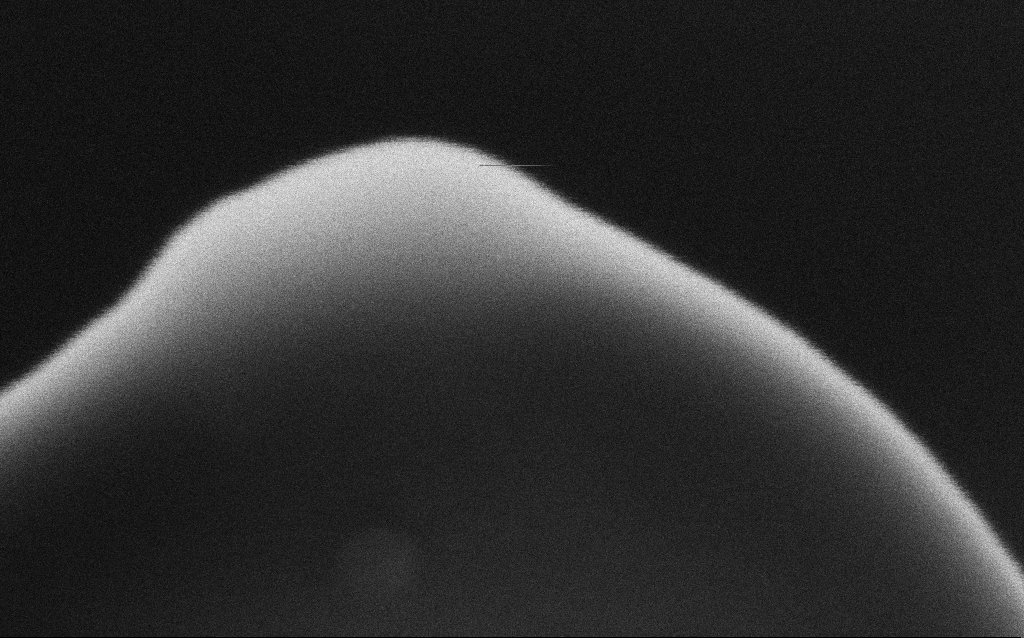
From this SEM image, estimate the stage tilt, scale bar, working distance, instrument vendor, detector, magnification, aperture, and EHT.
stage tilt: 0°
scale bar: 20 nm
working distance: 3.1 mm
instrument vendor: Zeiss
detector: InLens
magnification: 915.98 K X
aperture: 30 µm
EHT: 5 kV